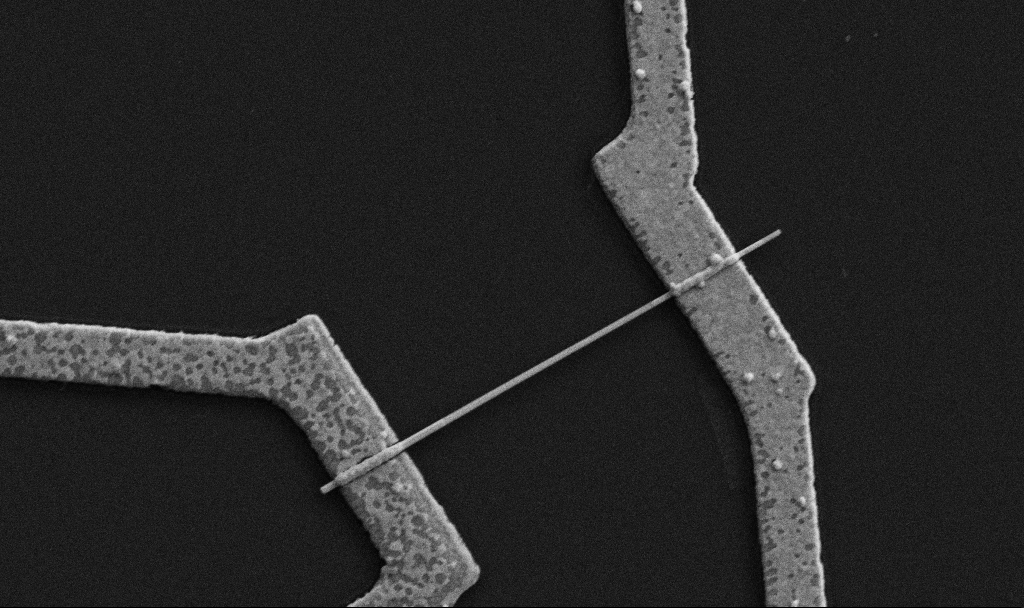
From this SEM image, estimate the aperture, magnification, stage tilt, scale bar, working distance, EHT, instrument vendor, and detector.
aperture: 30 µm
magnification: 30 K X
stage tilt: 0°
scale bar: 1000 nm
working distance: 8.7 mm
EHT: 5 kV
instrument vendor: Zeiss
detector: SE2